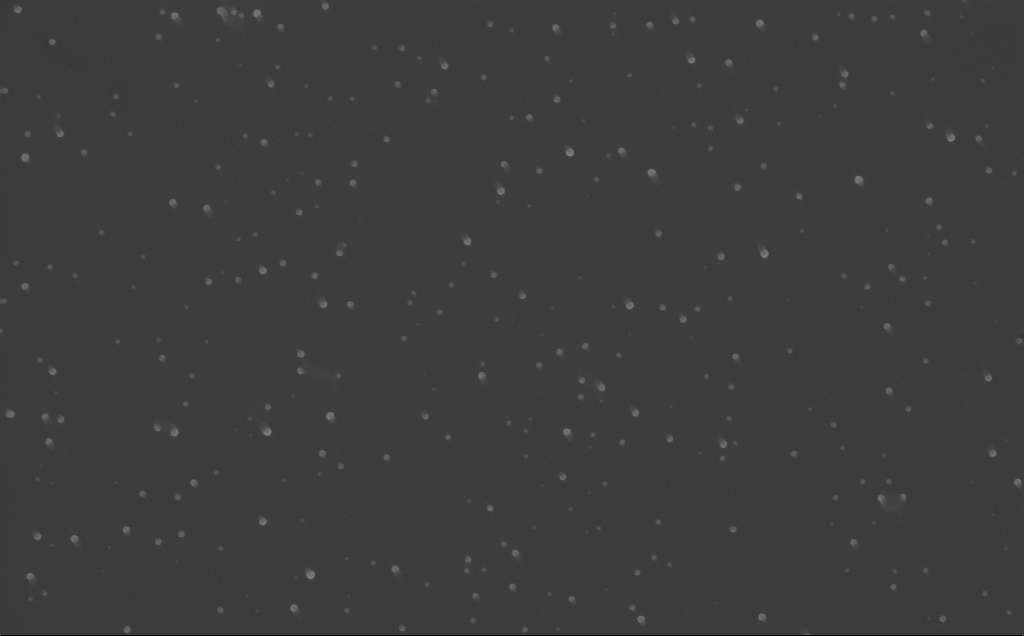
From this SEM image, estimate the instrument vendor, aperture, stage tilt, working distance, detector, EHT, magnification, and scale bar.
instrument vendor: Zeiss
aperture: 30 µm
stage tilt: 0°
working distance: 6 mm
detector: InLens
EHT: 10 kV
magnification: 40 K X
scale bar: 1000 nm